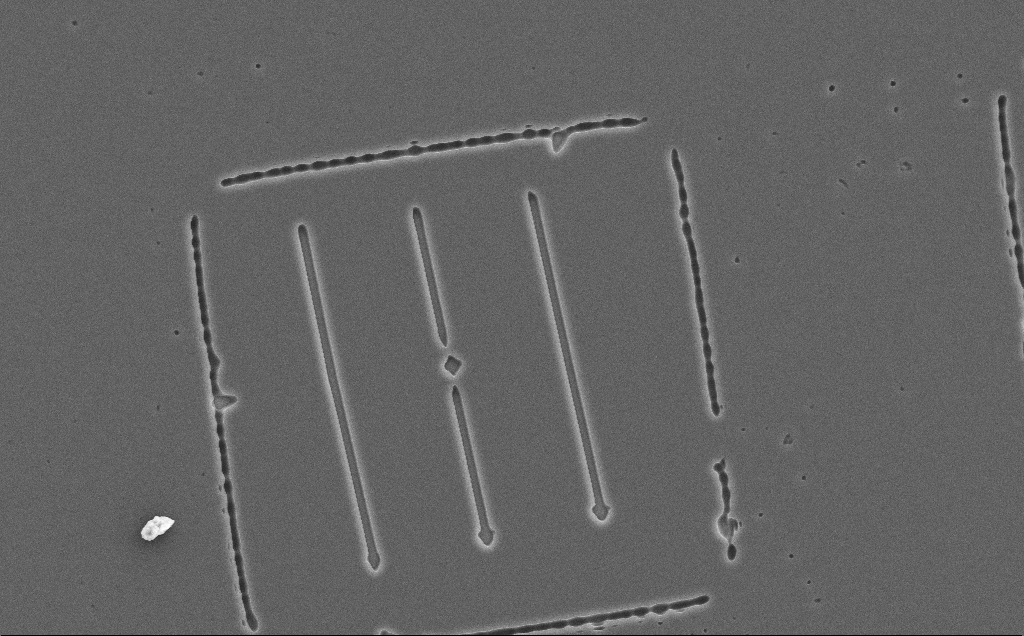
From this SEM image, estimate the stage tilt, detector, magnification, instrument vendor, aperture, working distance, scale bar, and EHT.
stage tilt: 0°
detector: SE2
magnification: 2.12 K X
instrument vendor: Zeiss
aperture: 30 µm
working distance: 12 mm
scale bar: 10000 nm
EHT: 10 kV